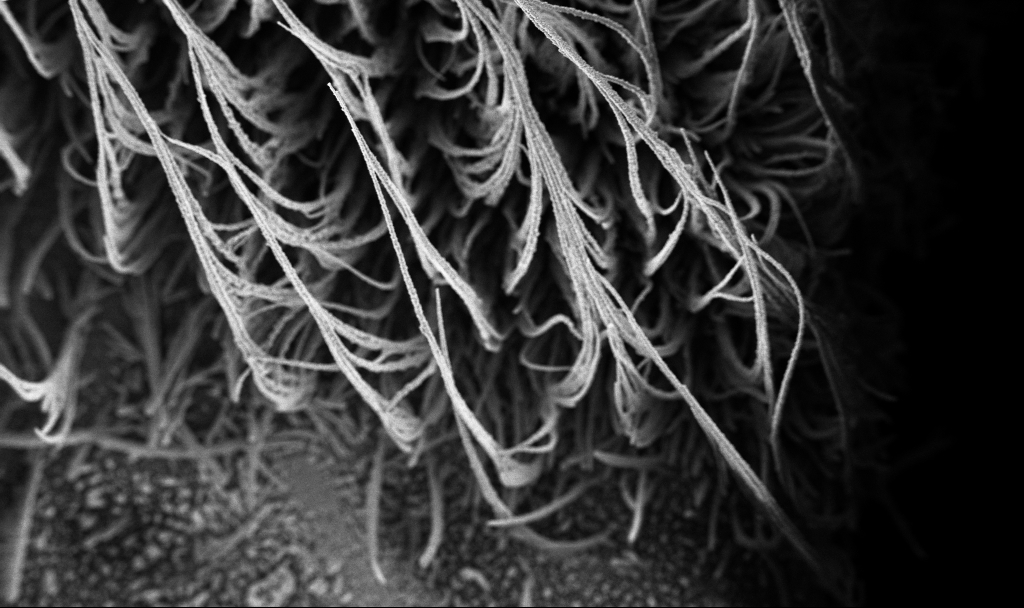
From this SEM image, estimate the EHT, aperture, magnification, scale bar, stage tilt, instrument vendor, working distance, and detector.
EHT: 3 kV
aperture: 30 µm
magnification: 0.15 K X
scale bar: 100000 nm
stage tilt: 0°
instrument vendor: Zeiss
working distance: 2.6 mm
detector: InLens